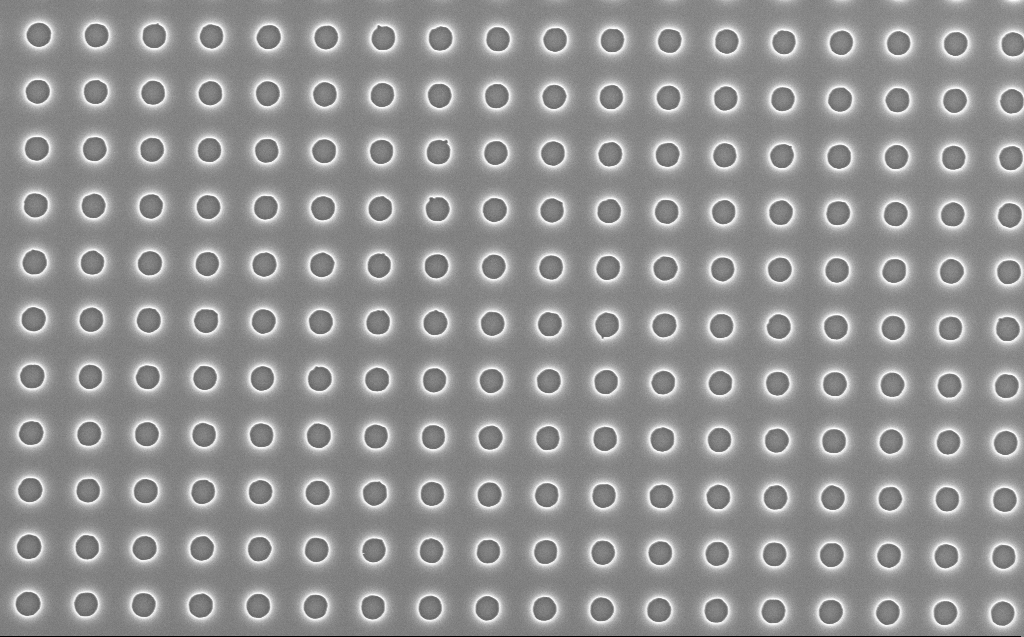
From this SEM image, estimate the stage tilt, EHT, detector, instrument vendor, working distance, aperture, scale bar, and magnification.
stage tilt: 0°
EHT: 5 kV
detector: InLens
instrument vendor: Zeiss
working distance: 7 mm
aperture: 30 µm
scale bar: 2000 nm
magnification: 20 K X